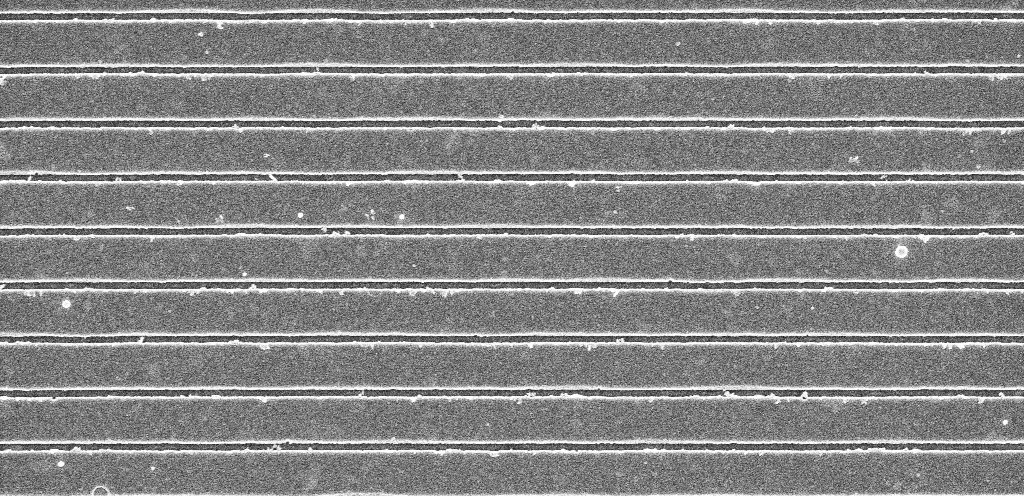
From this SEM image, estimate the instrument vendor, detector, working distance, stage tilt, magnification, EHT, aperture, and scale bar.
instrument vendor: Zeiss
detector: InLens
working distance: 5.2 mm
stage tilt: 0°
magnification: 28.43 K X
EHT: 5 kV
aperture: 30 µm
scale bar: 2000 nm